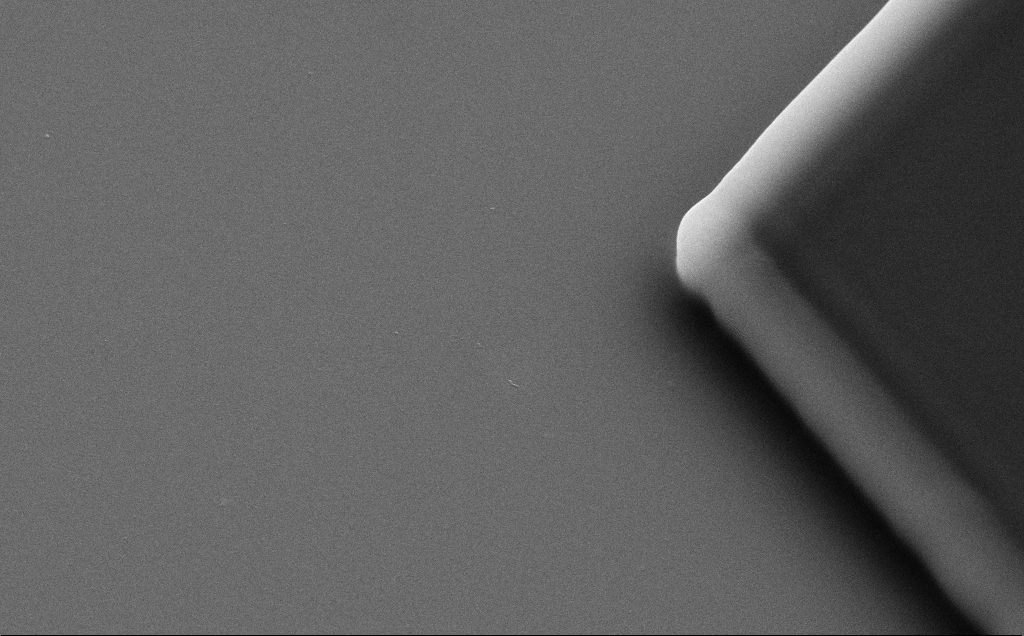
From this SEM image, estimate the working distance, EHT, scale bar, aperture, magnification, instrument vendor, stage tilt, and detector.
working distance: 8 mm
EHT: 10 kV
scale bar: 1000 nm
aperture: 30 µm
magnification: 19.8 K X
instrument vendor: Zeiss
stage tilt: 35°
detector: SE2